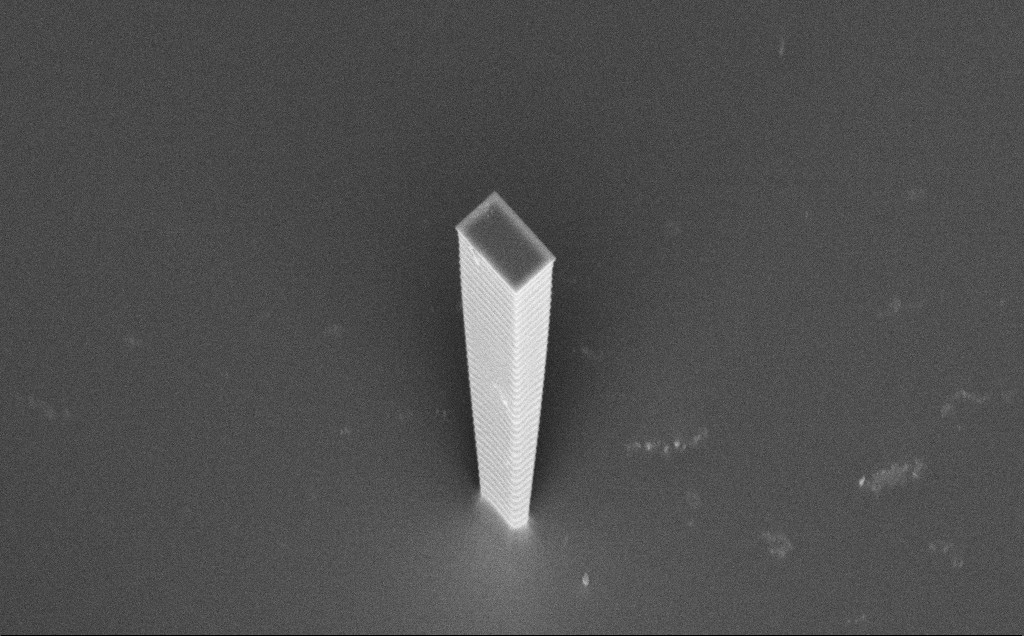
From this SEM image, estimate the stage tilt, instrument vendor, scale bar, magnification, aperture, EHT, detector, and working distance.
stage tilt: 45°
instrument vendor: Zeiss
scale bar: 10000 nm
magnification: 6.96 K X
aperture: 30 µm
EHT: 7.5 kV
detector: InLens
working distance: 8 mm